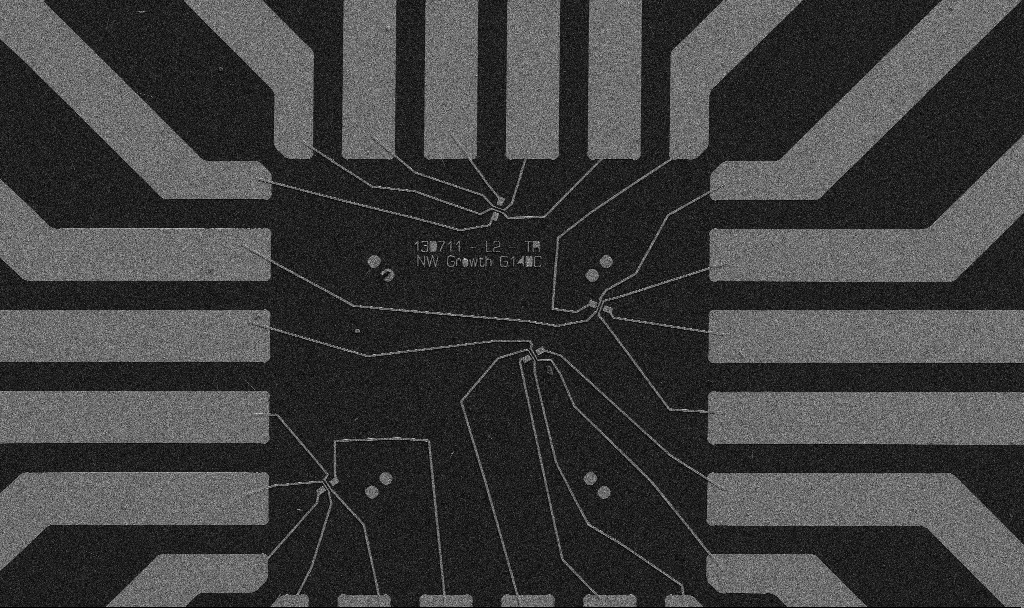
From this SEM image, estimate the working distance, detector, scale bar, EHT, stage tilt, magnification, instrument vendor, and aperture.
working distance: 10.7 mm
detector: SE2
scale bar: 20000 nm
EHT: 5 kV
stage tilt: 0°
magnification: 1 K X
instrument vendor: Zeiss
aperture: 30 µm